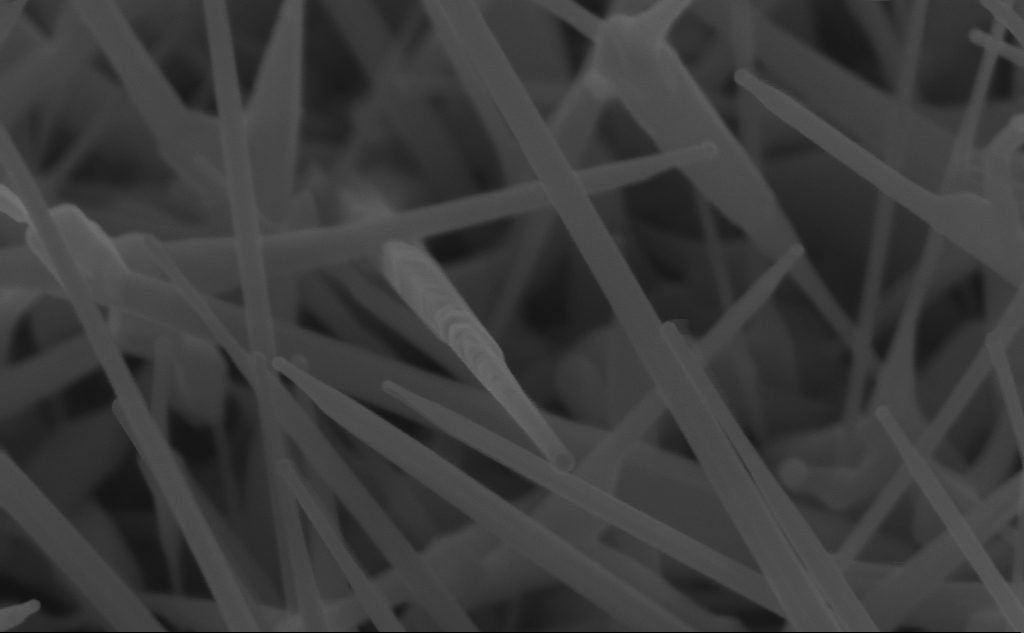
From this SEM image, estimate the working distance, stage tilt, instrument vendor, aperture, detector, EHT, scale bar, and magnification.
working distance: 5 mm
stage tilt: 45°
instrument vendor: Zeiss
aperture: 30 µm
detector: InLens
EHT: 10 kV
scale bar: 200 nm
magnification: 171.68 K X